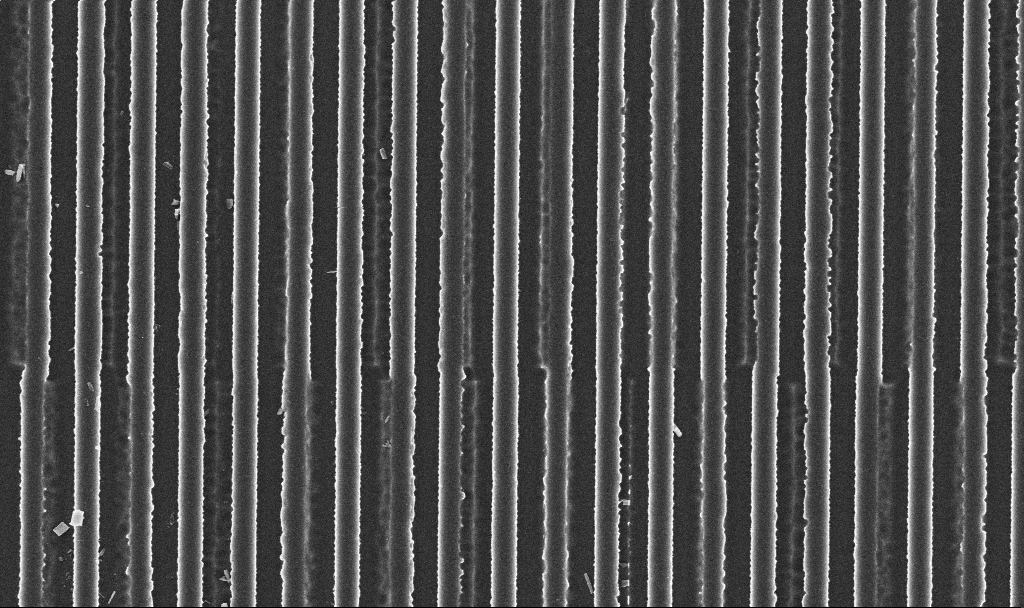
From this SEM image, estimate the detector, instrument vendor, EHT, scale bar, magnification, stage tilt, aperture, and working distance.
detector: InLens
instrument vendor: Zeiss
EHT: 3 kV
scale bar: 2000 nm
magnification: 29.21 K X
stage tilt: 0°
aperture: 30 µm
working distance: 2.9 mm